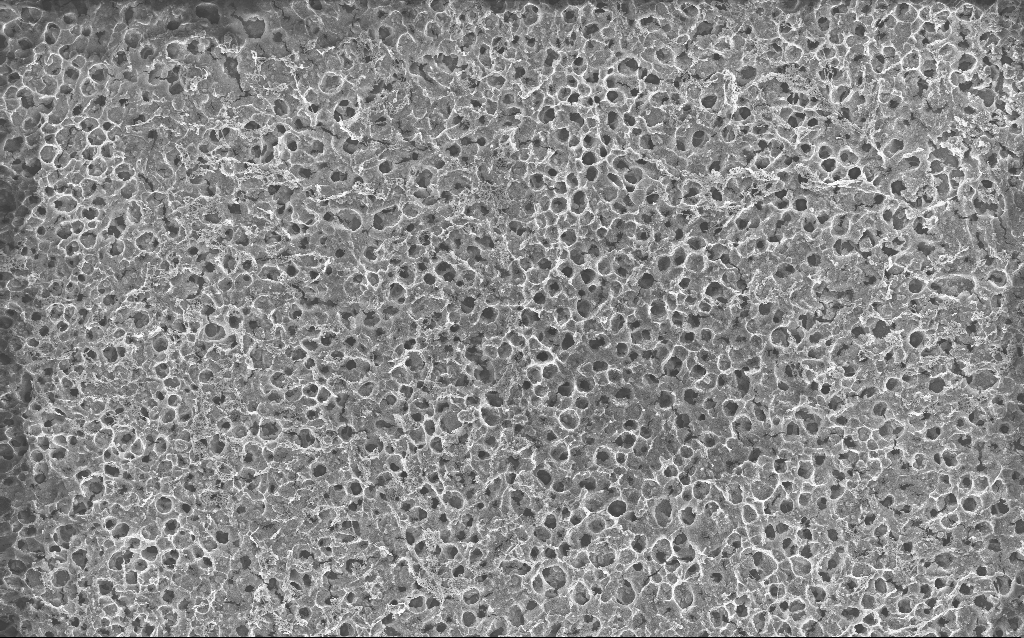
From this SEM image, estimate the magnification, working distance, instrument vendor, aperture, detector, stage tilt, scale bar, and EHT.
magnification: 0.792 K X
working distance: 2.4 mm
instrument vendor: Zeiss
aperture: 30 µm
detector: InLens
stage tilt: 0°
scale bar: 20000 nm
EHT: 10 kV